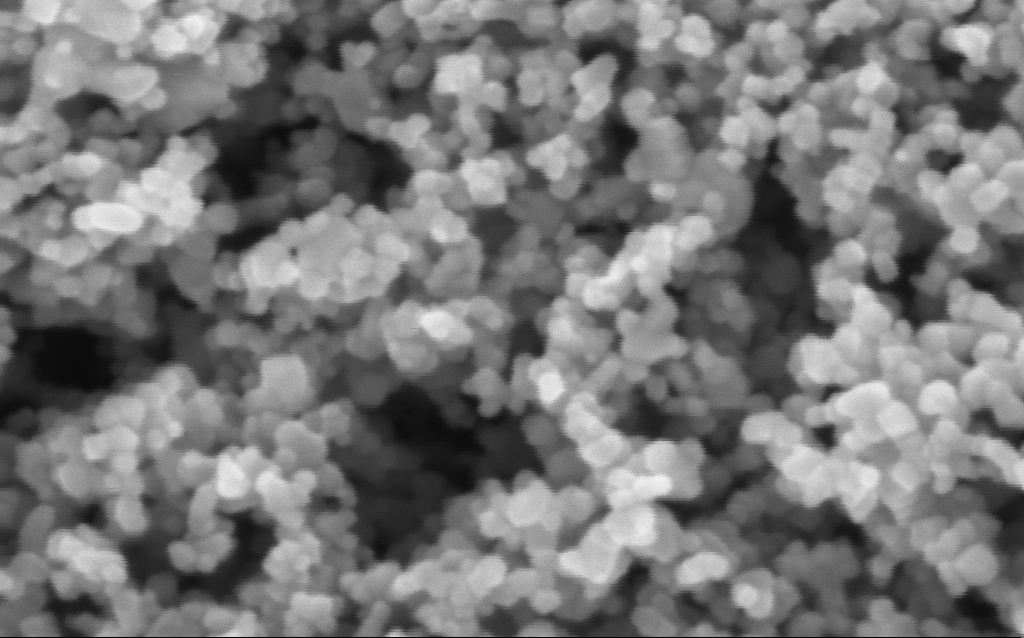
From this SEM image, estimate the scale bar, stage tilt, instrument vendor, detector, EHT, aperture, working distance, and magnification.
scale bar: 100 nm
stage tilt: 0°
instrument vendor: Zeiss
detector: InLens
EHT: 3 kV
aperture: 30 µm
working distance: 7.6 mm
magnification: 416 K X